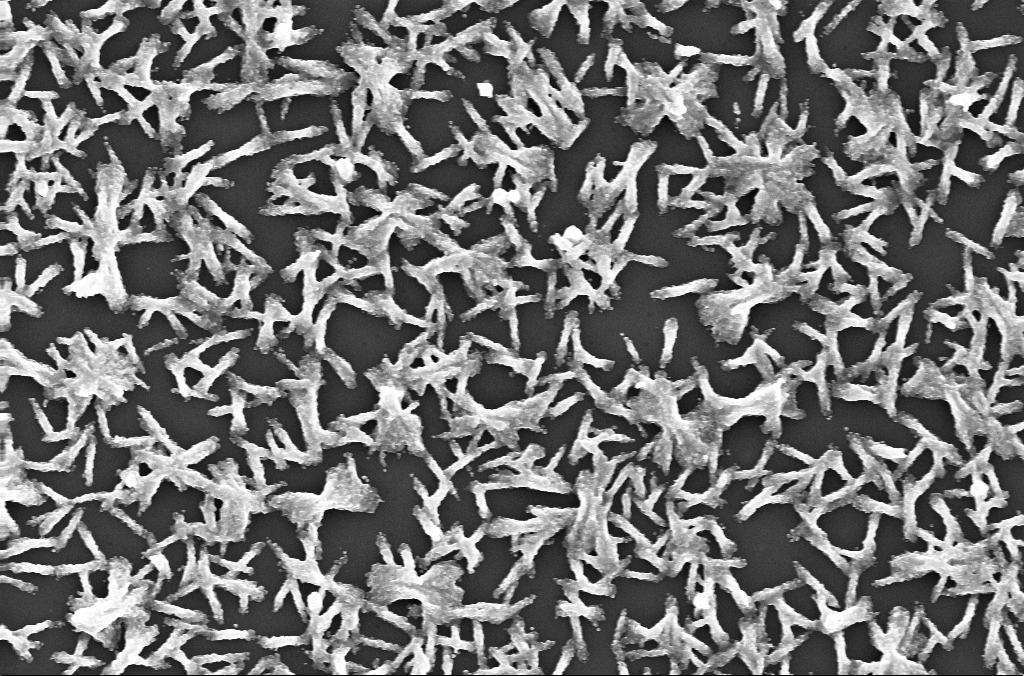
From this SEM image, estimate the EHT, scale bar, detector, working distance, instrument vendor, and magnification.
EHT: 20 kV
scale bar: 10000 nm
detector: InLens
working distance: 2.8 mm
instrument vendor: Zeiss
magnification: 5 K X